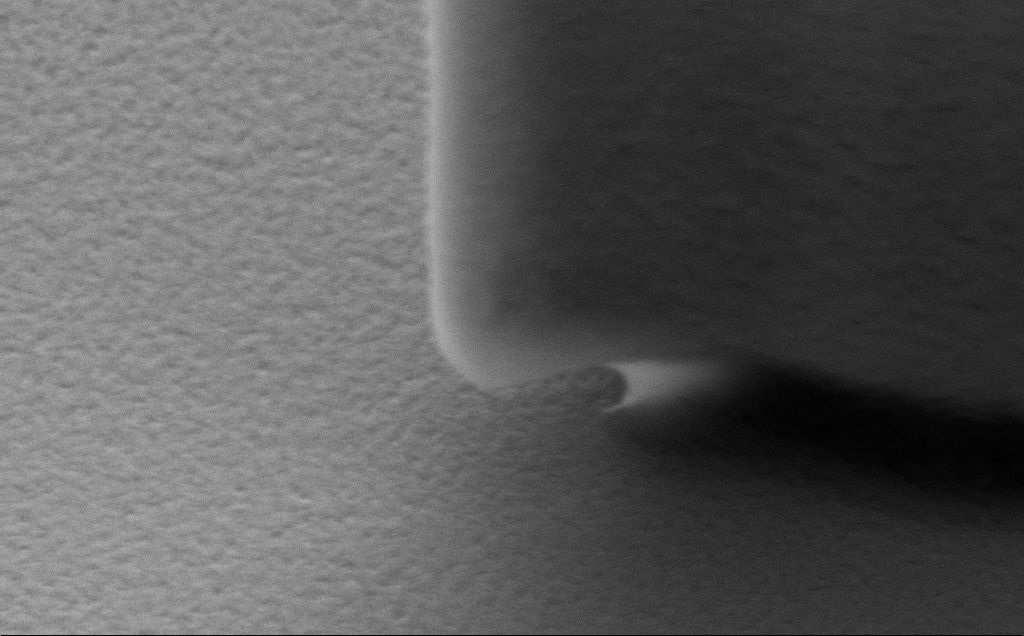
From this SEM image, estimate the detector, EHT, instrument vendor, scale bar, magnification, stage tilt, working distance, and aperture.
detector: SE2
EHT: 5 kV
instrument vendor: Zeiss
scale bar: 1000 nm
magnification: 57.76 K X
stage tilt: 40°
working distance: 9 mm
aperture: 30 µm